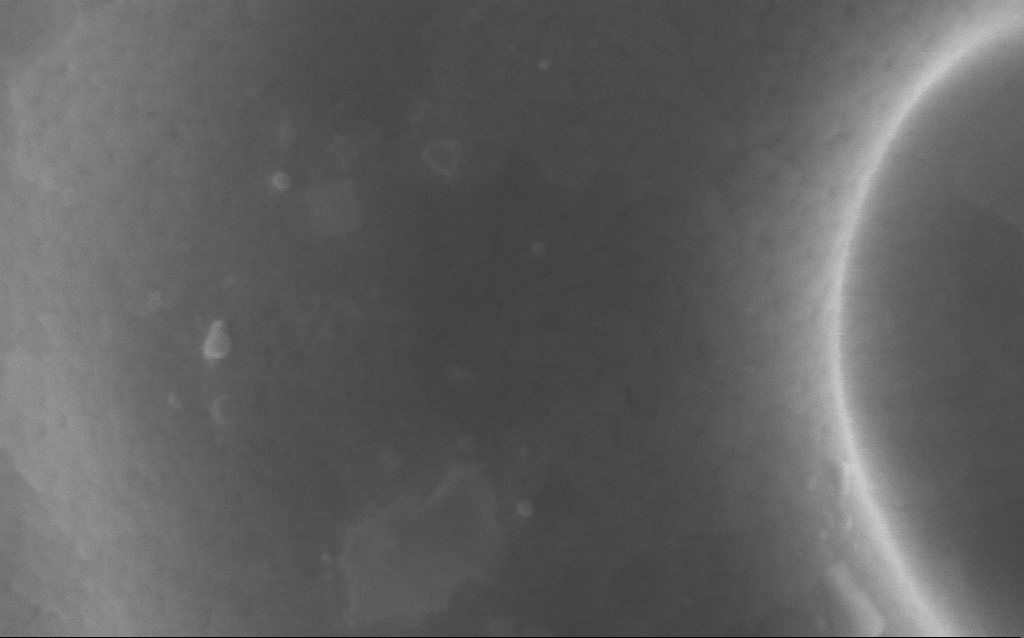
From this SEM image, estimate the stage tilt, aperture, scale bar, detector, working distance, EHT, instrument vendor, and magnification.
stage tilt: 0°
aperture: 30 µm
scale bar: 100 nm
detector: InLens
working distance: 4 mm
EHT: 5 kV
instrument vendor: Zeiss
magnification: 331 K X